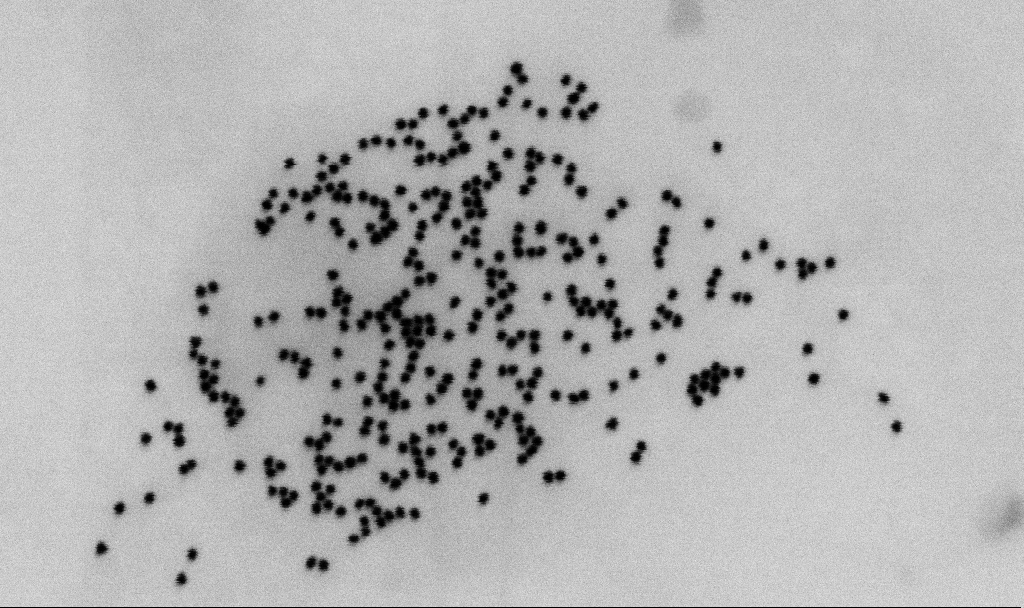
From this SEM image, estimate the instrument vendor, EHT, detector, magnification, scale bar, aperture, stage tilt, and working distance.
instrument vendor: Zeiss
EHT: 2 kV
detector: SE2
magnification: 181.5 K X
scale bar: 200 nm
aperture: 30 µm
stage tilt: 0°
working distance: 6.5 mm